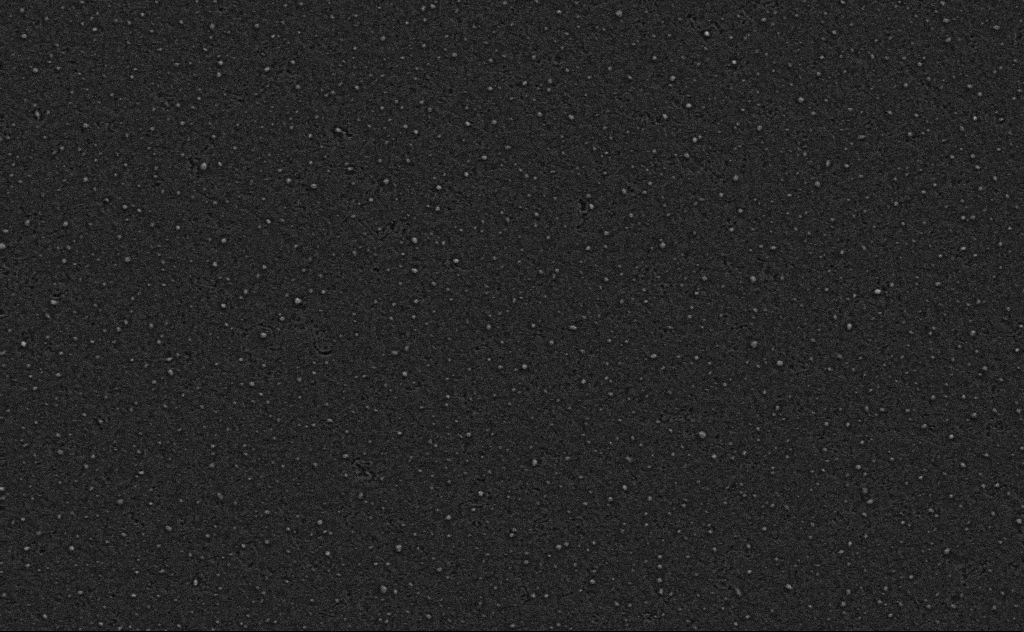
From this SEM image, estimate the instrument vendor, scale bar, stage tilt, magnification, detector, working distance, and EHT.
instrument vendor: Zeiss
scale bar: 1000 nm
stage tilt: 0°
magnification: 40 K X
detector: SE2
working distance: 4 mm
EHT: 3 kV